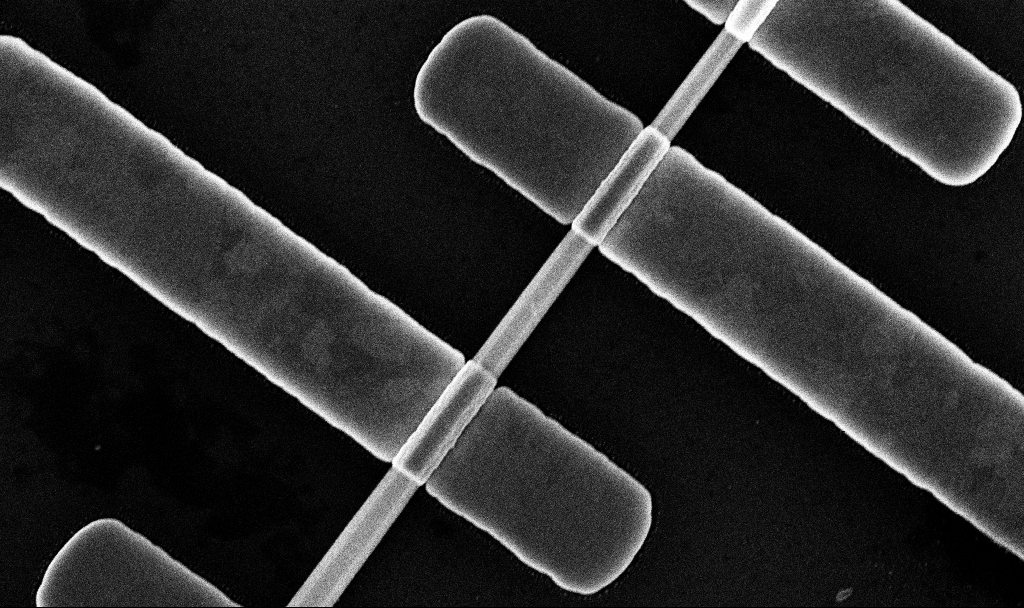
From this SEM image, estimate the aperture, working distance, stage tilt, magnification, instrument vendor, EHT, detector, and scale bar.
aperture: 30 µm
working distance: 7.8 mm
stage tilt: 0°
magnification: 66.4 K X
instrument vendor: Zeiss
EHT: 10 kV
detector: InLens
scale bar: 1000 nm